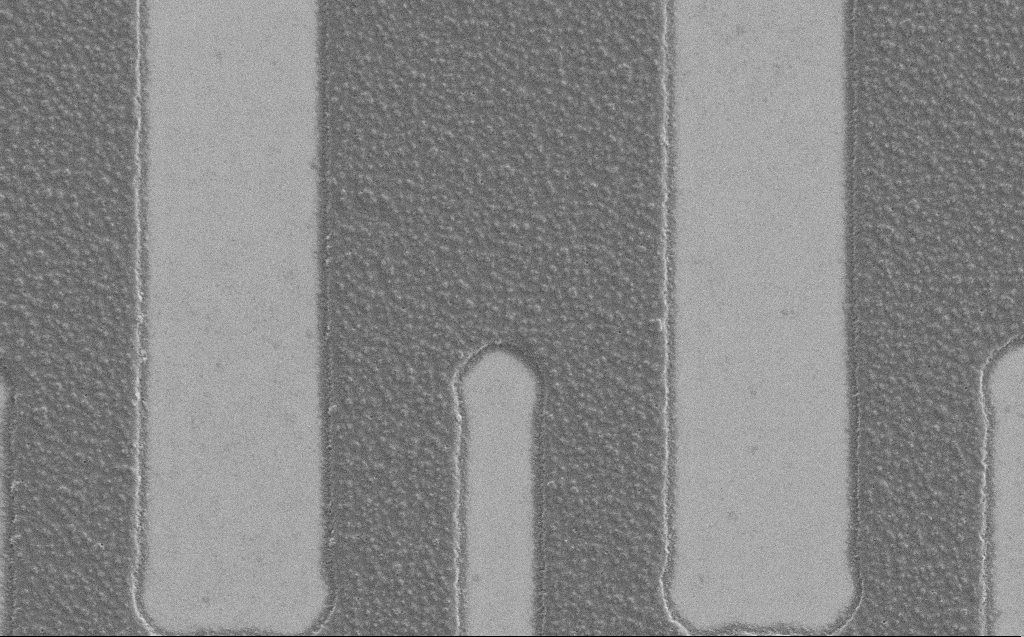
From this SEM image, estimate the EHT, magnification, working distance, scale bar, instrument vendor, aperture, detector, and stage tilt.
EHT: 1.2 kV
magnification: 7.07 K X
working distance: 4 mm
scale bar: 10000 nm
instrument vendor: Zeiss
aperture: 30 µm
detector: SE2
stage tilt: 0°